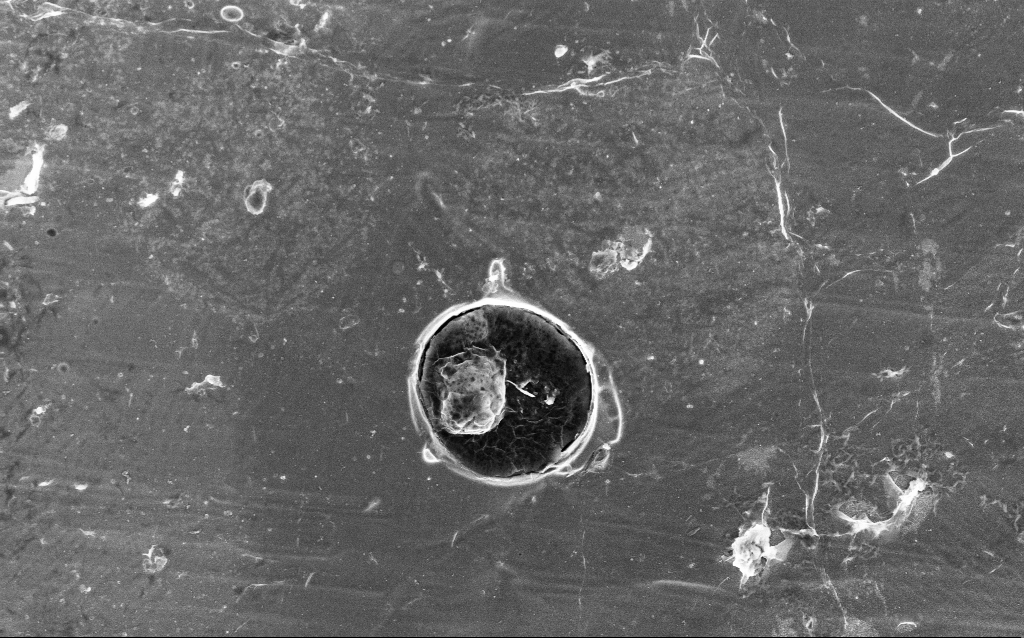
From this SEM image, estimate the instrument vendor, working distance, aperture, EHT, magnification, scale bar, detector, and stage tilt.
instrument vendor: Zeiss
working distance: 5.5 mm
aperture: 30 µm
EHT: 5 kV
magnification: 12.03 K X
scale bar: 1000 nm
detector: InLens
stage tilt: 0°